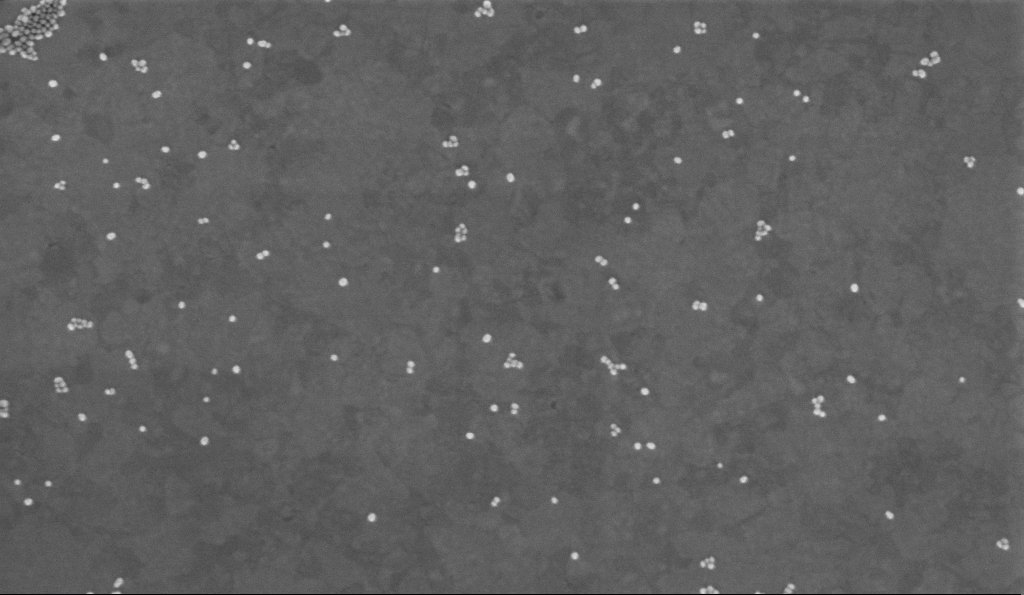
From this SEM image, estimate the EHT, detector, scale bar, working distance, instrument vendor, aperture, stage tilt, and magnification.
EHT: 2 kV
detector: InLens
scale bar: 200 nm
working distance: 3.4 mm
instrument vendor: Zeiss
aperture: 30 µm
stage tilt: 0°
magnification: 100 K X